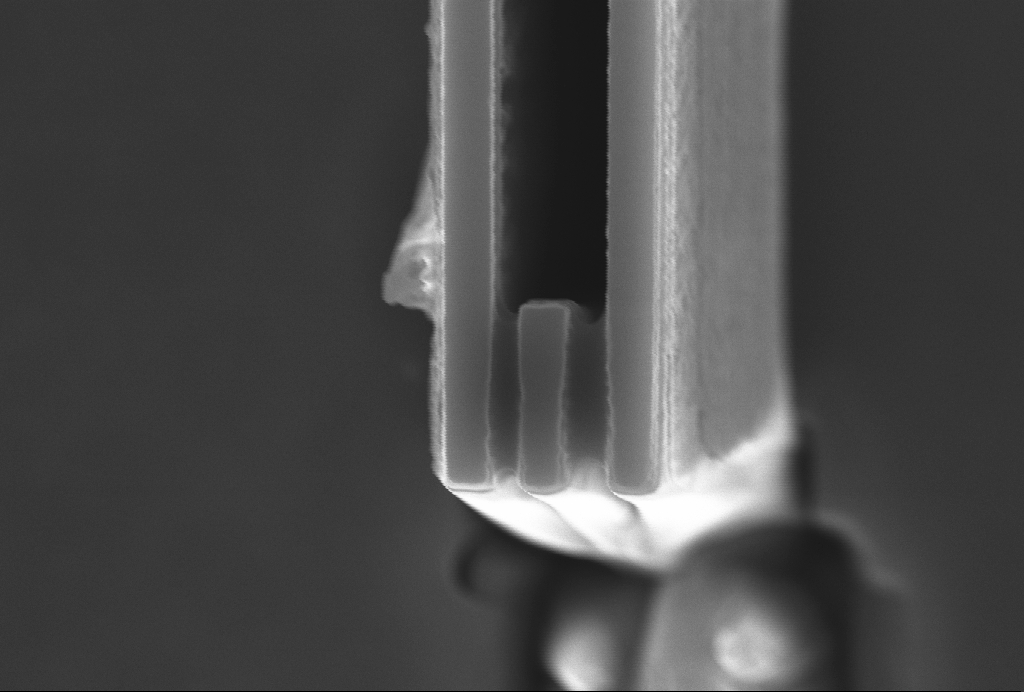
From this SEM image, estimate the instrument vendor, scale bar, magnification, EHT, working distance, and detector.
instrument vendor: Zeiss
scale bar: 1000 nm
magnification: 36.14 K X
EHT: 10 kV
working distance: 5 mm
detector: InLens